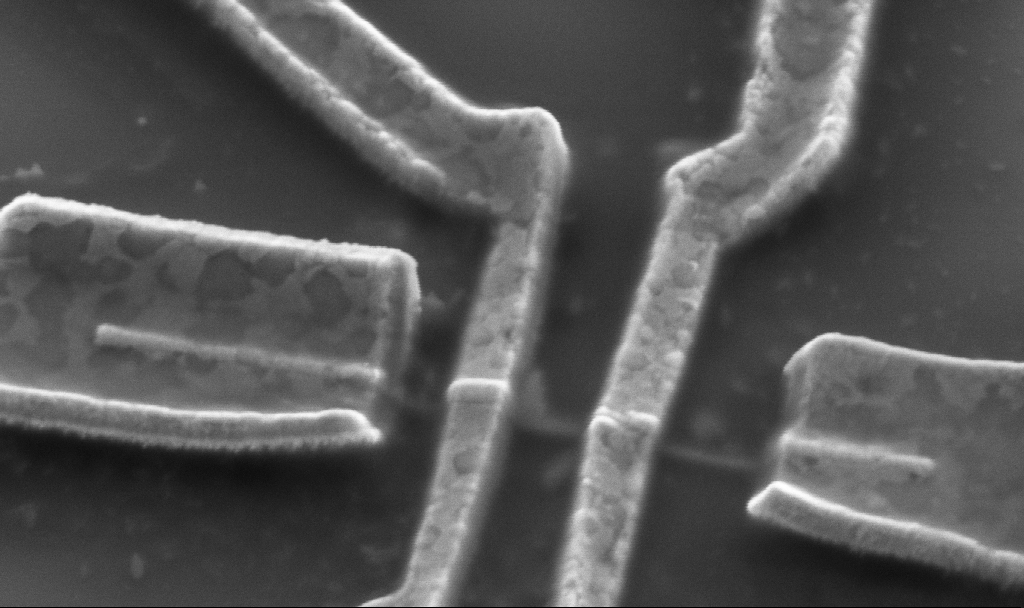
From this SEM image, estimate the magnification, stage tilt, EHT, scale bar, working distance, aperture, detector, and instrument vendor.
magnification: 50 K X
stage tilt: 45°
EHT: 5 kV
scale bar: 1000 nm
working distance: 16.7 mm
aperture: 30 µm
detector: SE2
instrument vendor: Zeiss